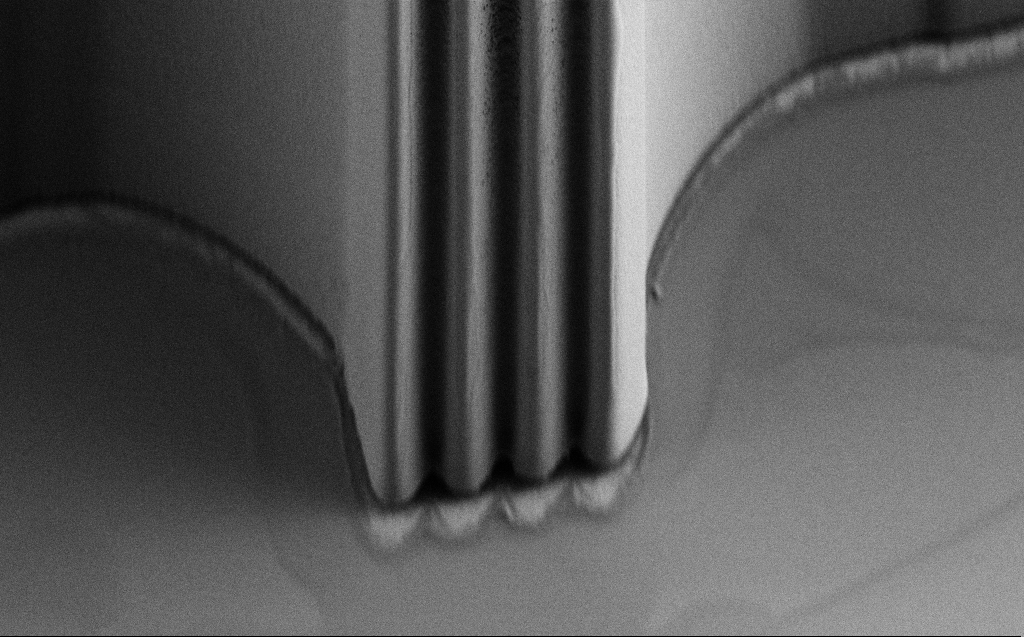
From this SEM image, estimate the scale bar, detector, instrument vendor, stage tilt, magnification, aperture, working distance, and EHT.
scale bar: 20000 nm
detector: SE2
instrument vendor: Zeiss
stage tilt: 45°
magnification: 2.31 K X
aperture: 30 µm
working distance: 6 mm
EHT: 0.9 kV